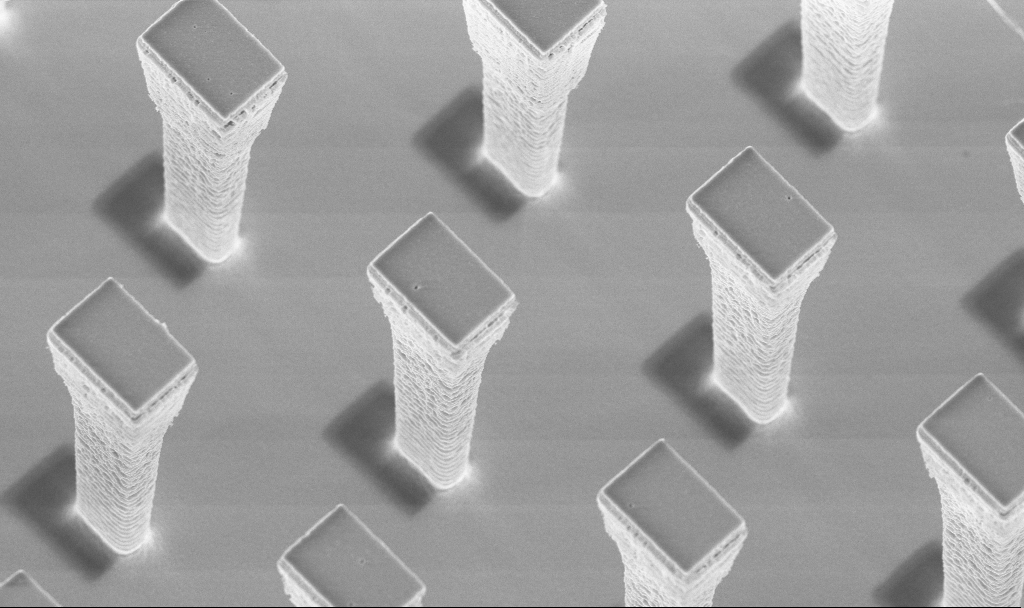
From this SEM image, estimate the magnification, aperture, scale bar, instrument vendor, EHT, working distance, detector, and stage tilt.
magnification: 10.25 K X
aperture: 30 µm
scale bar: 2000 nm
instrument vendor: Zeiss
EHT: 5 kV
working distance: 4.2 mm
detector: InLens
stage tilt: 20°